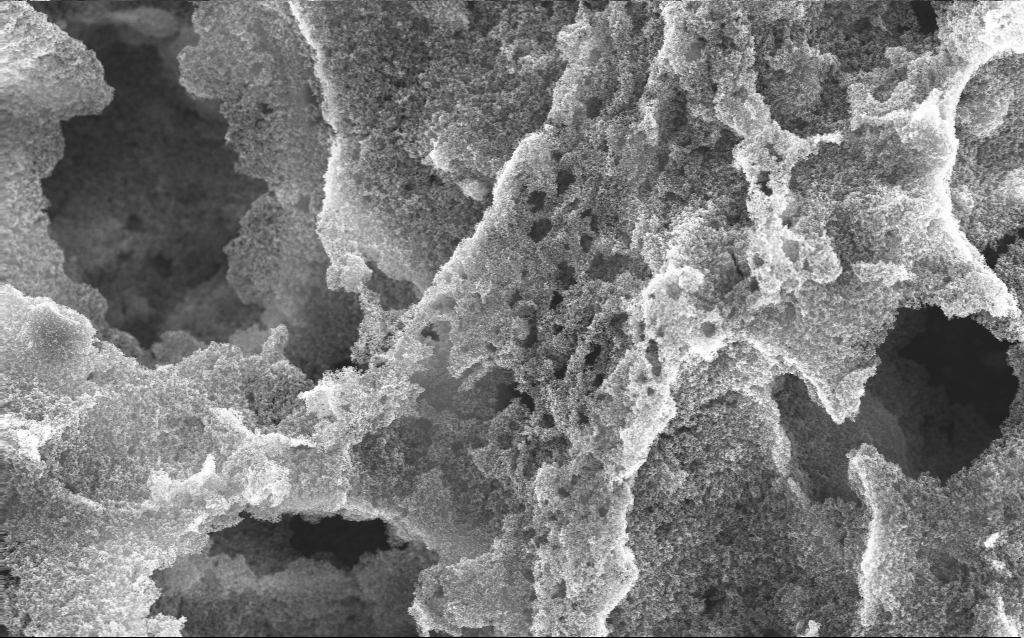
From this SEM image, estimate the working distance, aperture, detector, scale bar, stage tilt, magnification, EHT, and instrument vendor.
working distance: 2.4 mm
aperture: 30 µm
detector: InLens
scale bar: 1000 nm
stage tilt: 0°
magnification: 15.33 K X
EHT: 10 kV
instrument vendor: Zeiss